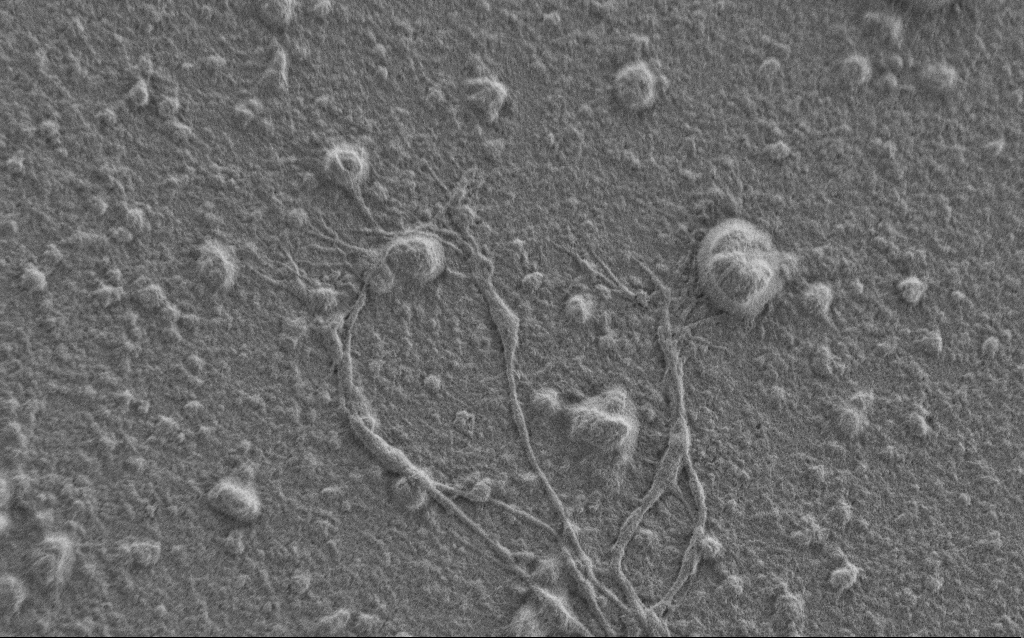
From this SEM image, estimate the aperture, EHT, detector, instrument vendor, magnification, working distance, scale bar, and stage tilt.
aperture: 30 µm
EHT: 0.9 kV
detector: SE2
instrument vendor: Zeiss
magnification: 5 K X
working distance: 7 mm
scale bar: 10000 nm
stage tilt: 0°